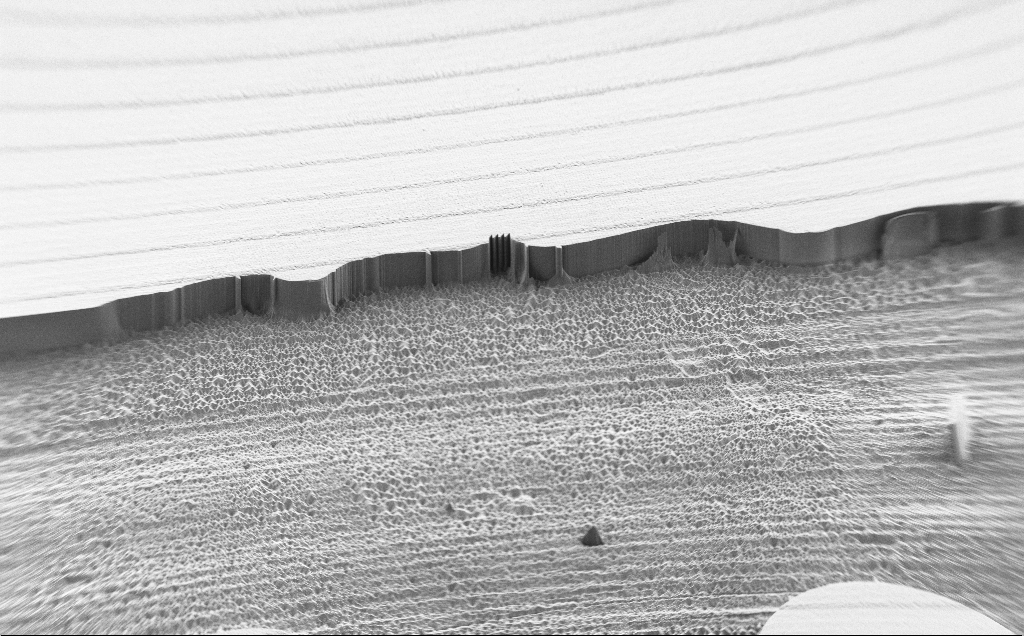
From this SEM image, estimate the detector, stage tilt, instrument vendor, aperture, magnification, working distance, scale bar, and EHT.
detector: SE2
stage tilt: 45°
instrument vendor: Zeiss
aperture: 30 µm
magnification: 0.19 K X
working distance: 7 mm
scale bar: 200000 nm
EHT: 1.2 kV